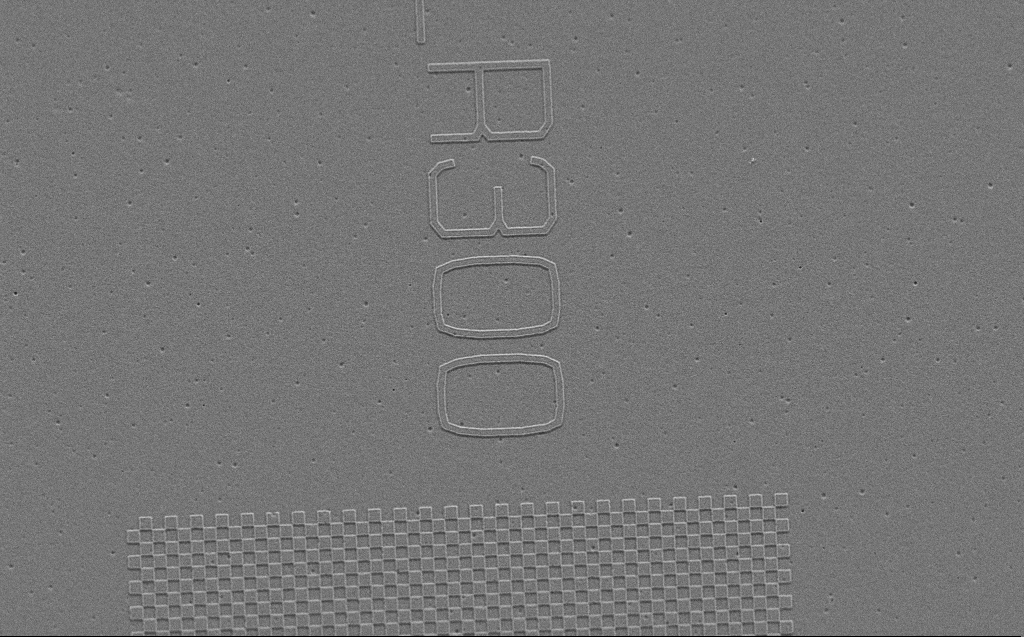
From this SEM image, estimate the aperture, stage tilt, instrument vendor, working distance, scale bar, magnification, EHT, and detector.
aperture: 30 µm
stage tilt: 29.8°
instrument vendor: Zeiss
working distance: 4 mm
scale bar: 2000 nm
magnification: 9.52 K X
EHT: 2 kV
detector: SE2